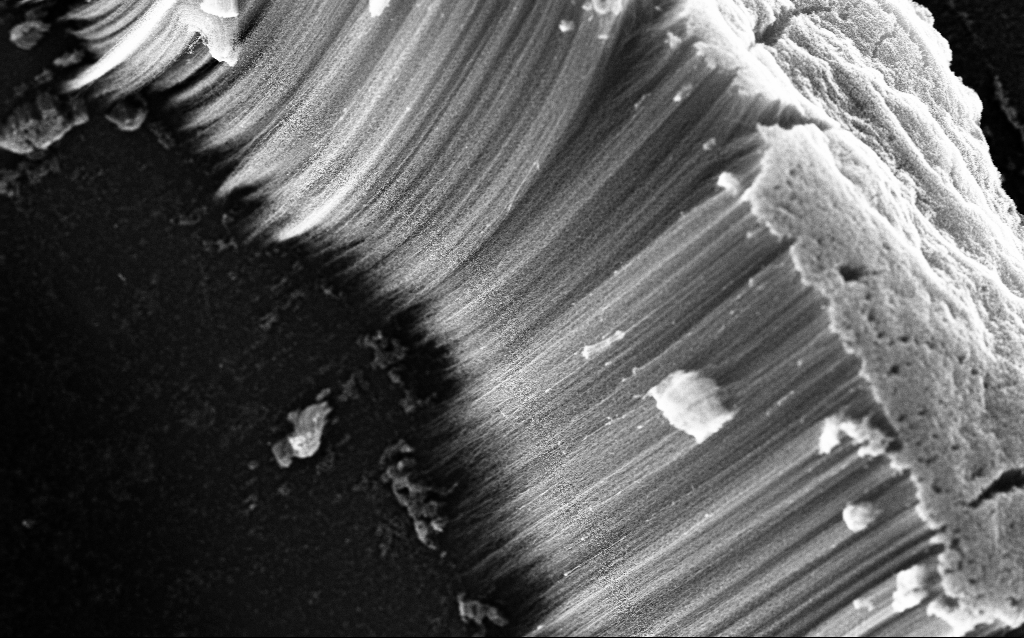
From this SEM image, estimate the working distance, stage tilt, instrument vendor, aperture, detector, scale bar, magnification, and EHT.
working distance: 2.2 mm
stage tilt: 0°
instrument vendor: Zeiss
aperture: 30 µm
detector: InLens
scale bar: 10000 nm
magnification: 1.87 K X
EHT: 5 kV